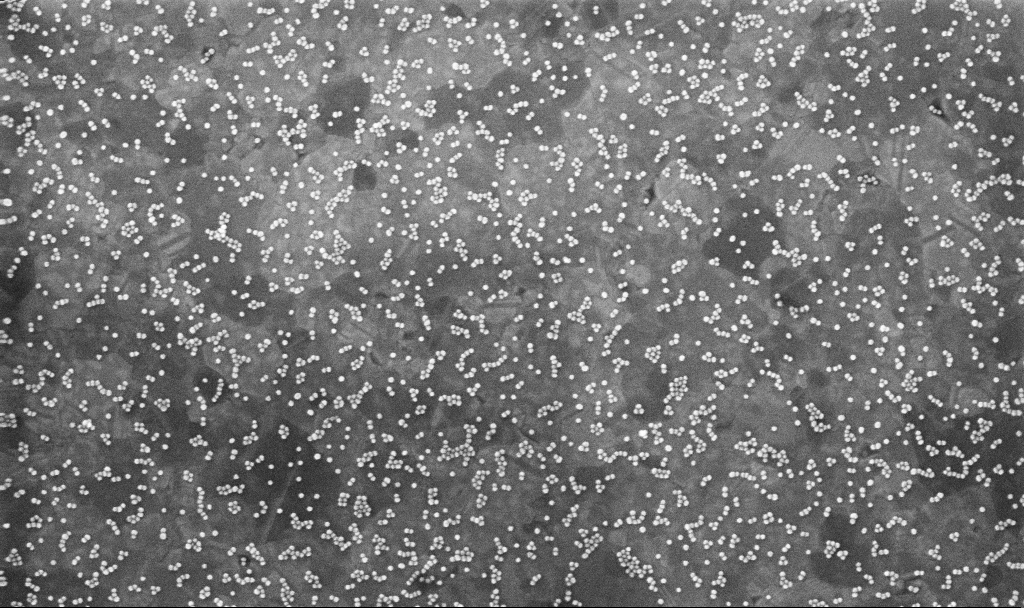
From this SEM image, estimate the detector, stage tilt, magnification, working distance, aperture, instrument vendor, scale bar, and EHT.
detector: InLens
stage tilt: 0°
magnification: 100 K X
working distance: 3.8 mm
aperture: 30 µm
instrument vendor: Zeiss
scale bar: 200 nm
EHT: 10 kV